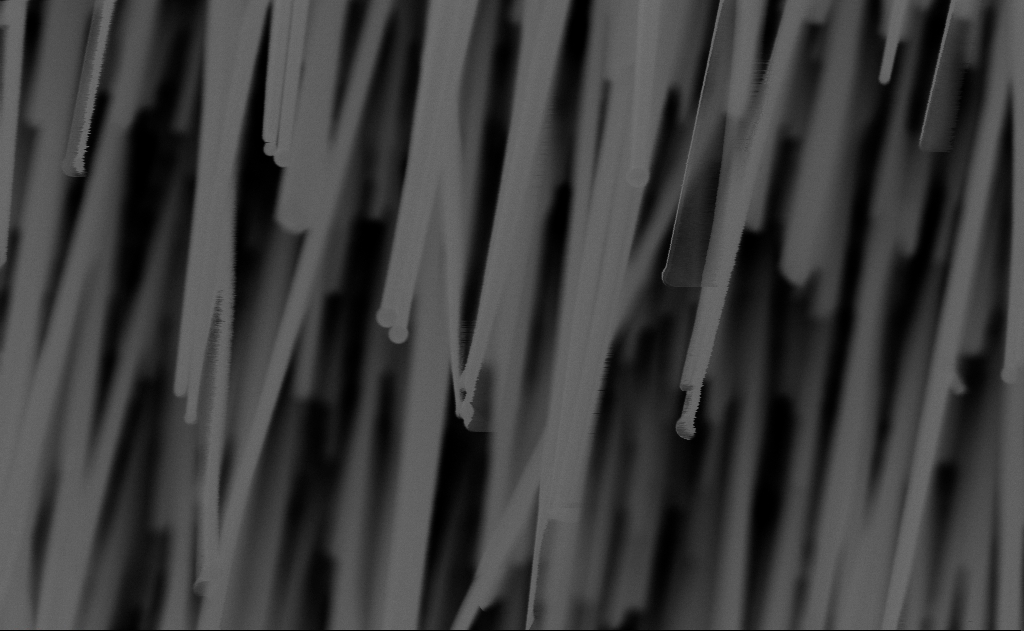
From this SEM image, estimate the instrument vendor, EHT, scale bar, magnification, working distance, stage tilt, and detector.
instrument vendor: Zeiss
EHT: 10 kV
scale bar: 200 nm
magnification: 80 K X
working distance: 9 mm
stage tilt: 0°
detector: InLens